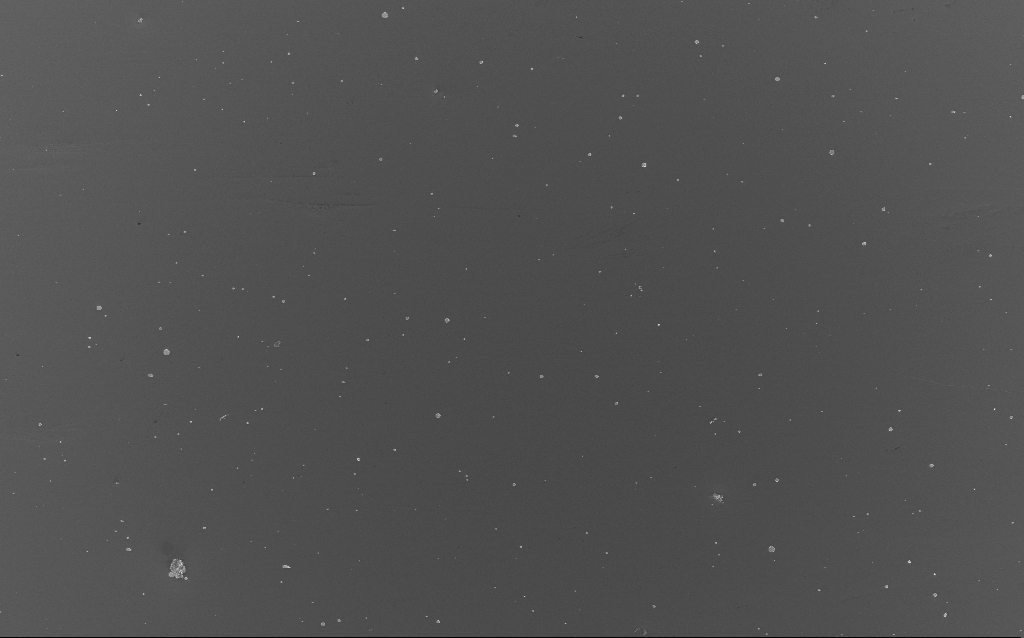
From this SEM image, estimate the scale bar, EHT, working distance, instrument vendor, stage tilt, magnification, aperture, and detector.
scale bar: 20000 nm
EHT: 3 kV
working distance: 4 mm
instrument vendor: Zeiss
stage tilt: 0°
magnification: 0.737 K X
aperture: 30 µm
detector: InLens